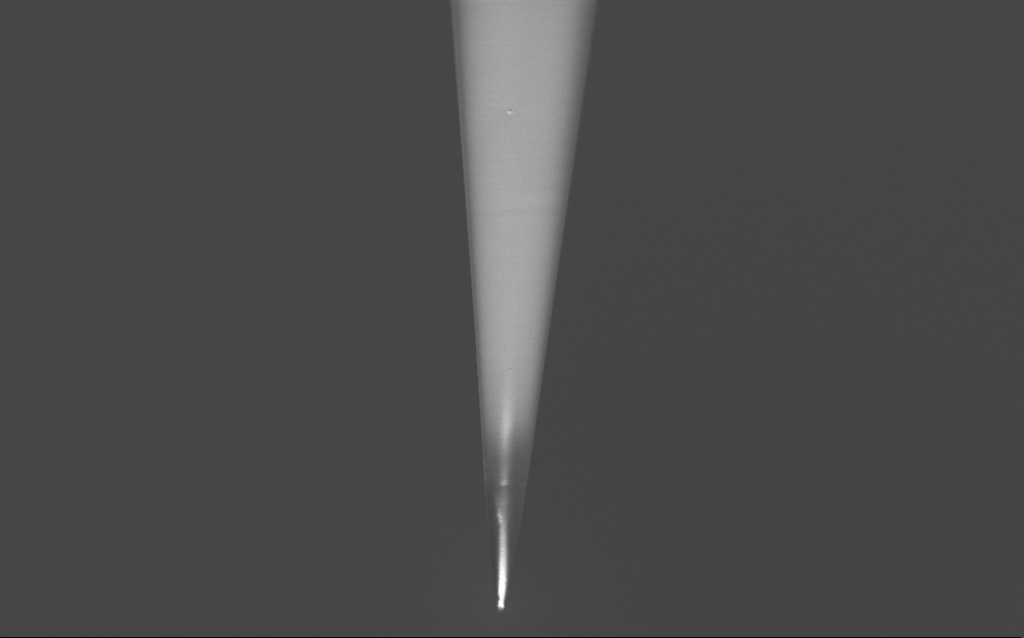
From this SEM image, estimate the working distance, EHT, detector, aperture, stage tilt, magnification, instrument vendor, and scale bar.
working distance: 6 mm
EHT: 2 kV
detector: InLens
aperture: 30 µm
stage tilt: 45°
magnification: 5 K X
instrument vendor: Zeiss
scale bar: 10000 nm